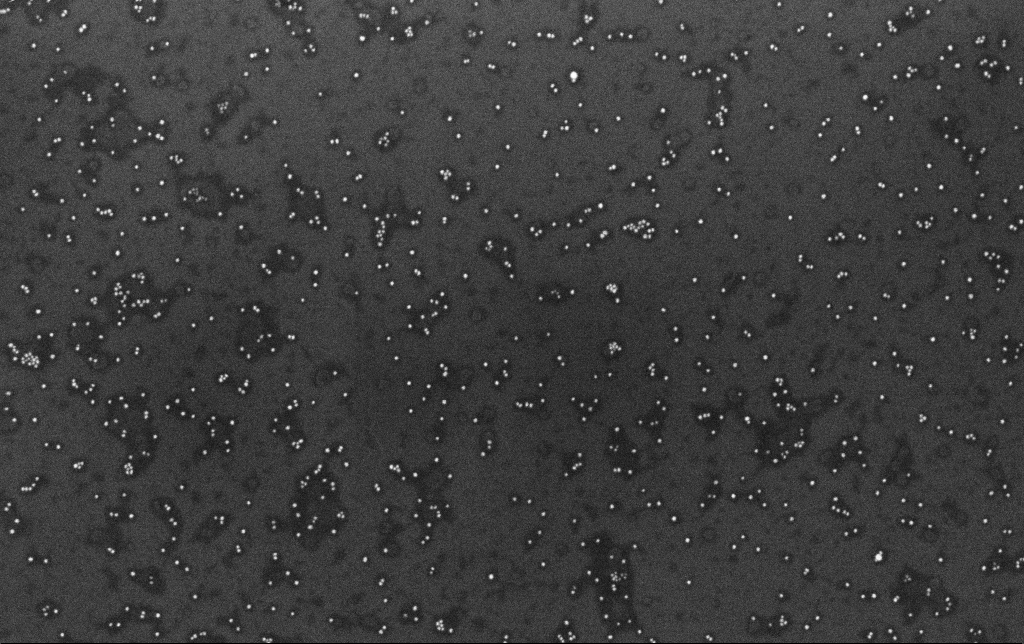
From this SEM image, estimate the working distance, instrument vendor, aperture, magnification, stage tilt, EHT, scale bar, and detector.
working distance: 3.4 mm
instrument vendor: Zeiss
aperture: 30 µm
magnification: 100 K X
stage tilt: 0°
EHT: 10 kV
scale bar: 200 nm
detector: InLens